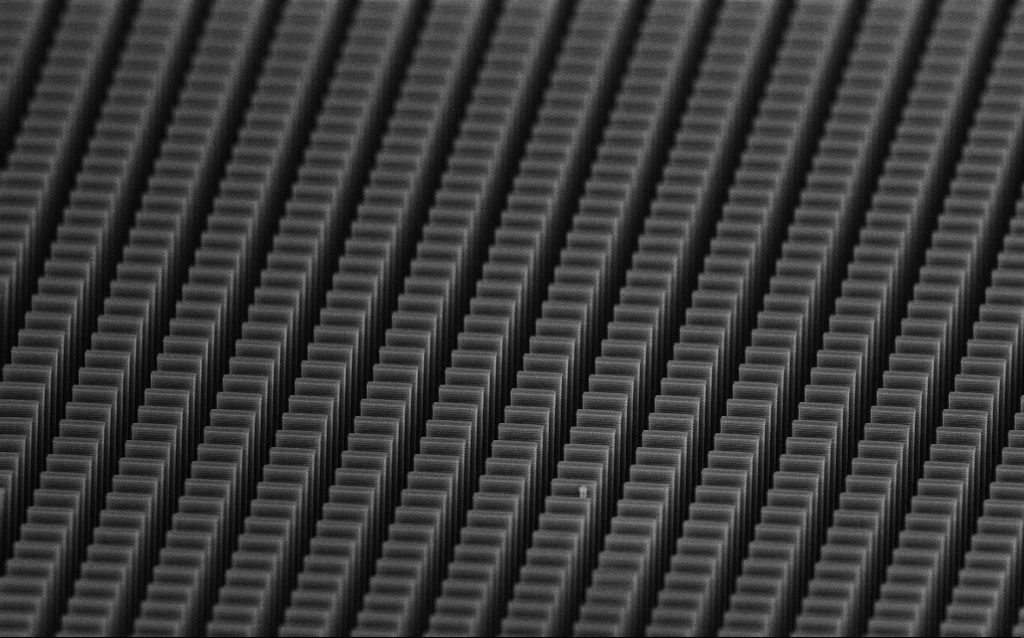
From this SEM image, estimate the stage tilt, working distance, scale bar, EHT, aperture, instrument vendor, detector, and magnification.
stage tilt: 70°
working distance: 4.4 mm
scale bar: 10000 nm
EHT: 10 kV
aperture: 30 µm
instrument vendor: Zeiss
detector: InLens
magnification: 3.39 K X